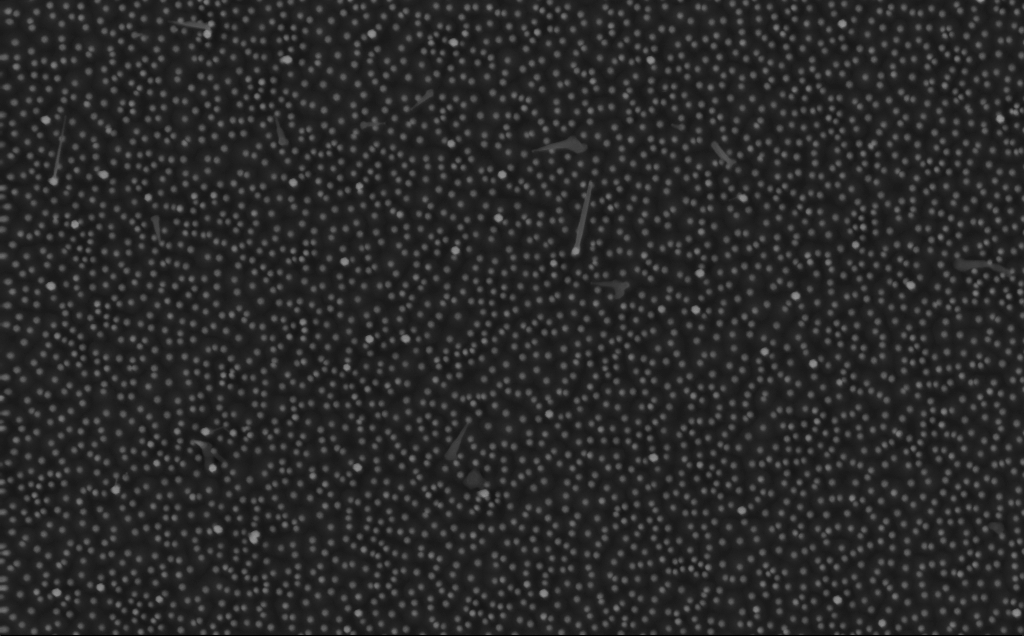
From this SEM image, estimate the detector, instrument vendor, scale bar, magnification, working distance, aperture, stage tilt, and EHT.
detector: InLens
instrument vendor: Zeiss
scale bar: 1000 nm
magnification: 40 K X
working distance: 4 mm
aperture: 30 µm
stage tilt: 0°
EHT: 10 kV